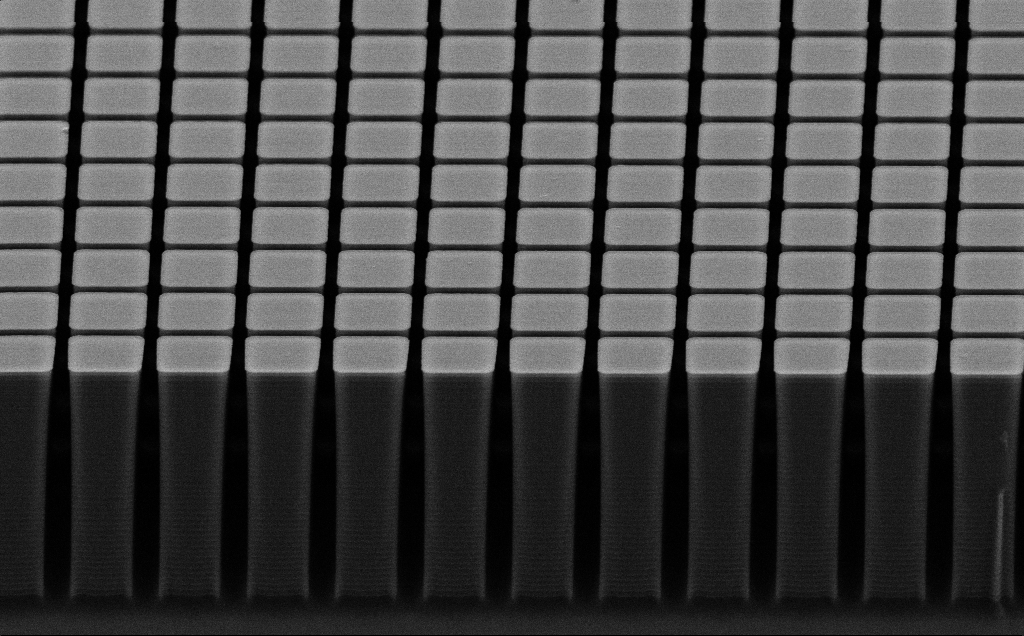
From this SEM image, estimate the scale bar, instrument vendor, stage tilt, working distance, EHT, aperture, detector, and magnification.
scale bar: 10000 nm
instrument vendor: Zeiss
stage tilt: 60.9°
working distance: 8 mm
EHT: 10 kV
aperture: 30 µm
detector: SE2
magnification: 6.54 K X